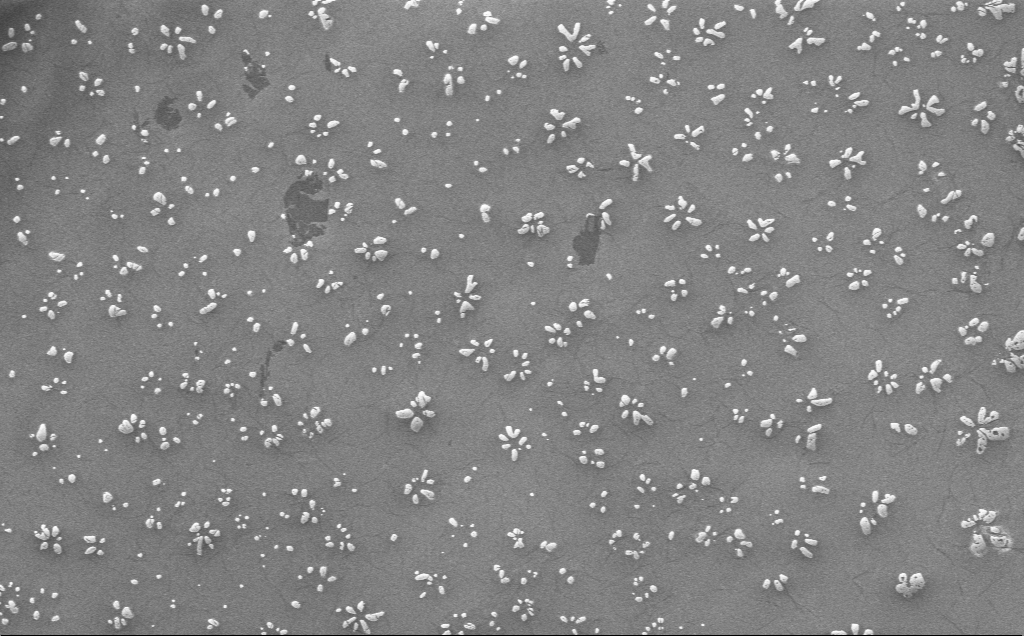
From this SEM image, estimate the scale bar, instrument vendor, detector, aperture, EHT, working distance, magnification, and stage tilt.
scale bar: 10000 nm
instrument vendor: Zeiss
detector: InLens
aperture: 30 µm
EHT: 1 kV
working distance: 4 mm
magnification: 1.56 K X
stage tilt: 0°